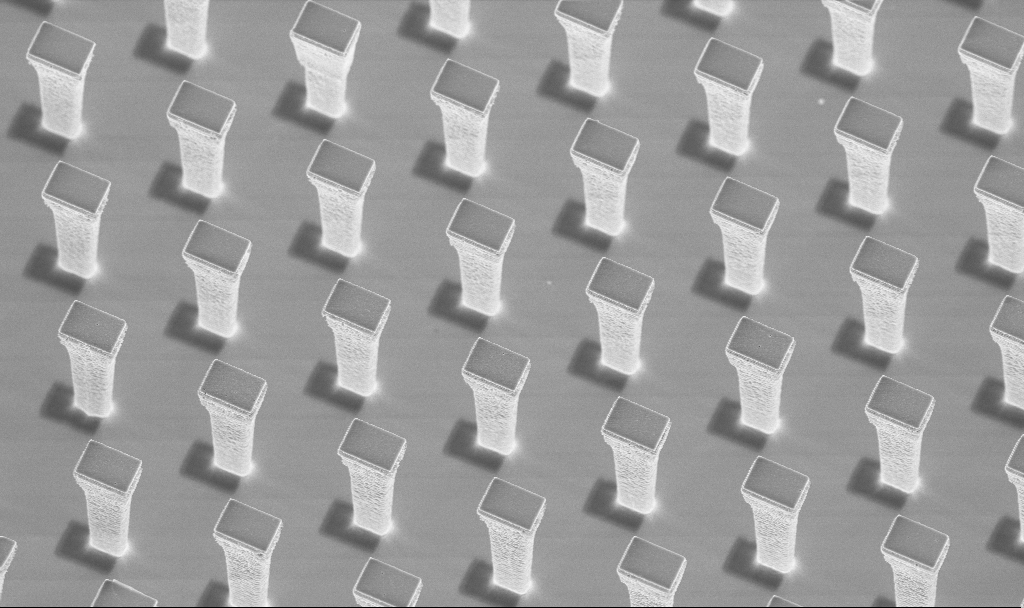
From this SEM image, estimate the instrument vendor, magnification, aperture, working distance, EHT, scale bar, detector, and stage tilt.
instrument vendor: Zeiss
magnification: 4.72 K X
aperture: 30 µm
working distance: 4.3 mm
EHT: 5 kV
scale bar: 10000 nm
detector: InLens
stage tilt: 20°